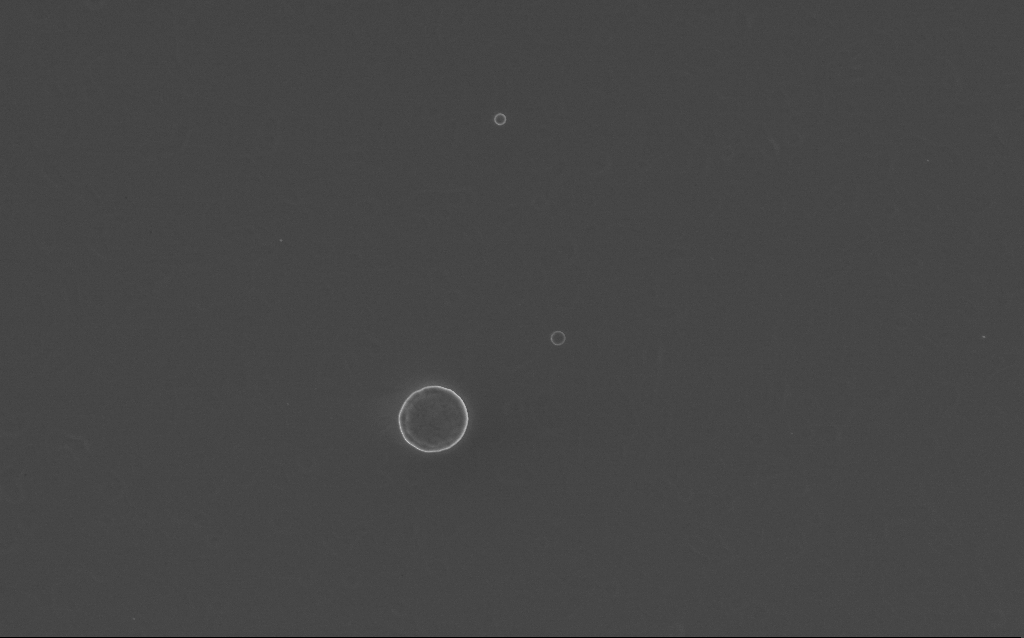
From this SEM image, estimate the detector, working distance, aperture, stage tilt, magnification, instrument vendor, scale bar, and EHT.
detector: InLens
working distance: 4 mm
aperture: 30 µm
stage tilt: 0°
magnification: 6.51 K X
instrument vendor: Zeiss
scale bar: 10000 nm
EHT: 10 kV